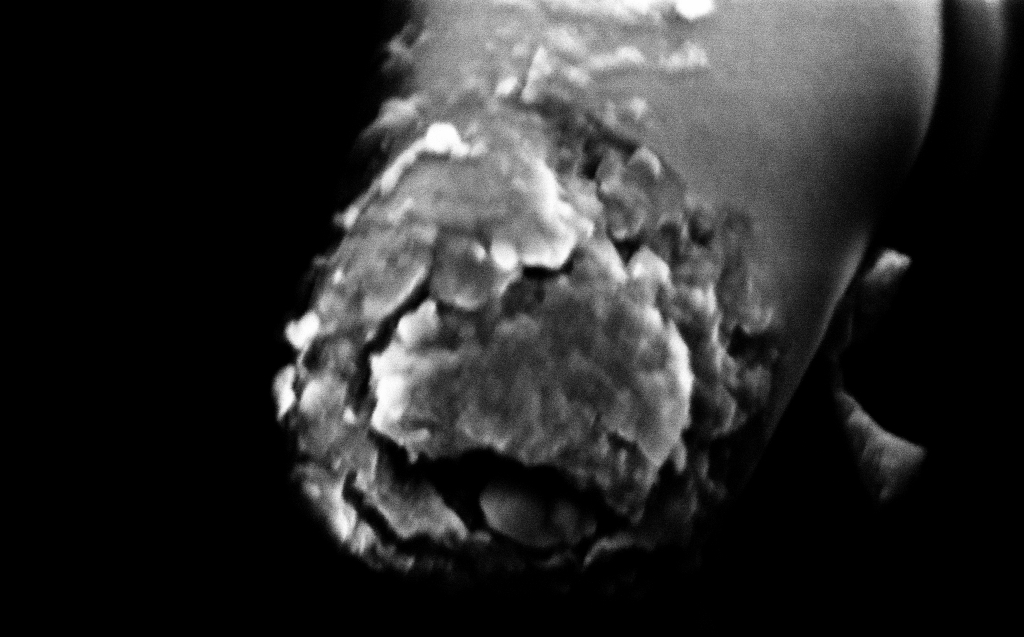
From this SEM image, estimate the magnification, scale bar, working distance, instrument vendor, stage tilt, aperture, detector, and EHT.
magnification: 200 K X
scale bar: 200 nm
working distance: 5 mm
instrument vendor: Zeiss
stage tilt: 45°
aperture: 30 µm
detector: InLens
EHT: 2 kV